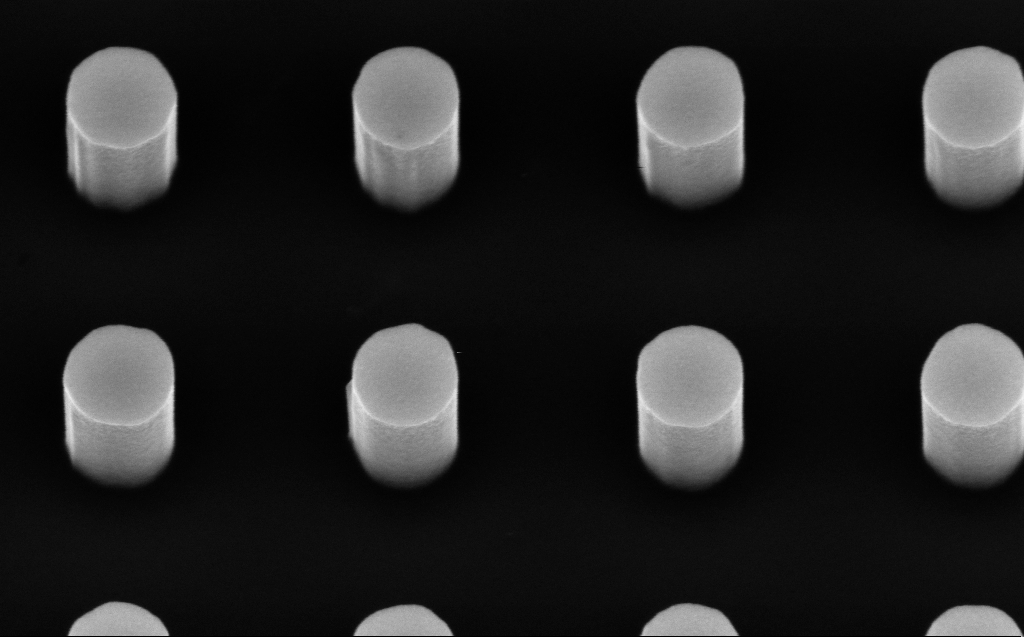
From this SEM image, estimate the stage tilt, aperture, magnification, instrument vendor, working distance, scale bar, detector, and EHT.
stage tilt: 30°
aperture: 30 µm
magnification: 100 K X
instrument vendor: Zeiss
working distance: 6 mm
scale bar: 200 nm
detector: InLens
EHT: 5 kV